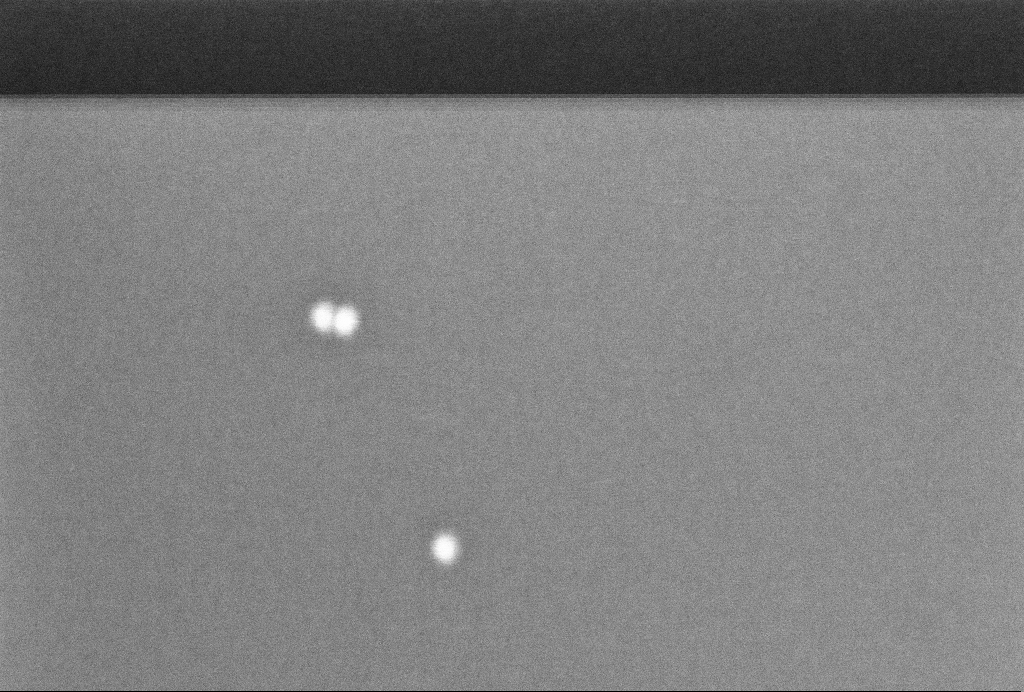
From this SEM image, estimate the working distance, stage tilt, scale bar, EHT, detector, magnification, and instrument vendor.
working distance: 3.2 mm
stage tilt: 0°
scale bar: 100 nm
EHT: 4 kV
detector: InLens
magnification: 350 K X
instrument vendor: Zeiss